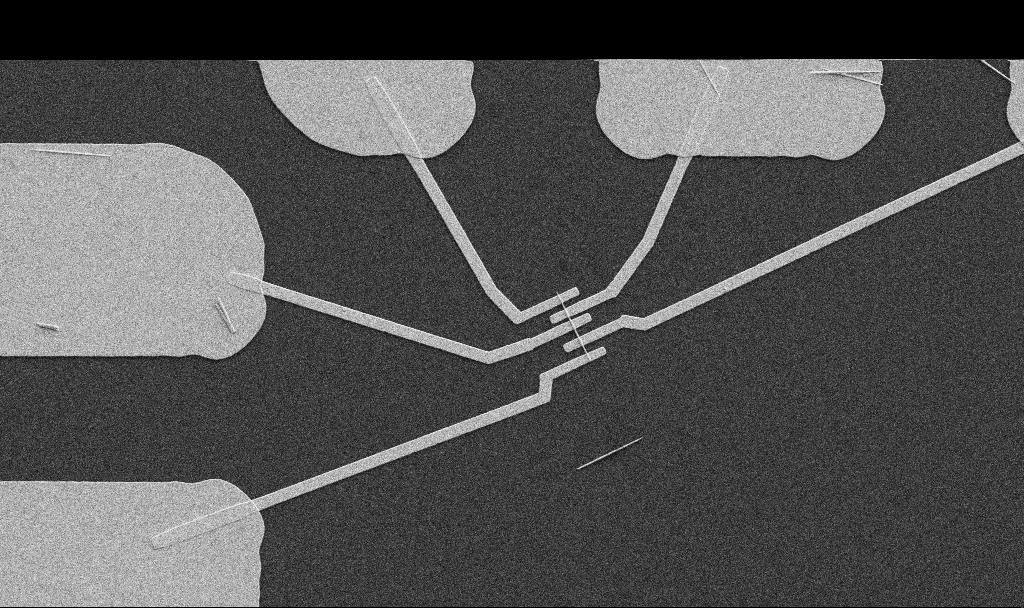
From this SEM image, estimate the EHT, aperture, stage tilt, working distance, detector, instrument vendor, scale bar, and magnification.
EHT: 5 kV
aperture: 30 µm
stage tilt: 0°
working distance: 10.7 mm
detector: SE2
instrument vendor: Zeiss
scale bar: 10000 nm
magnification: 5 K X